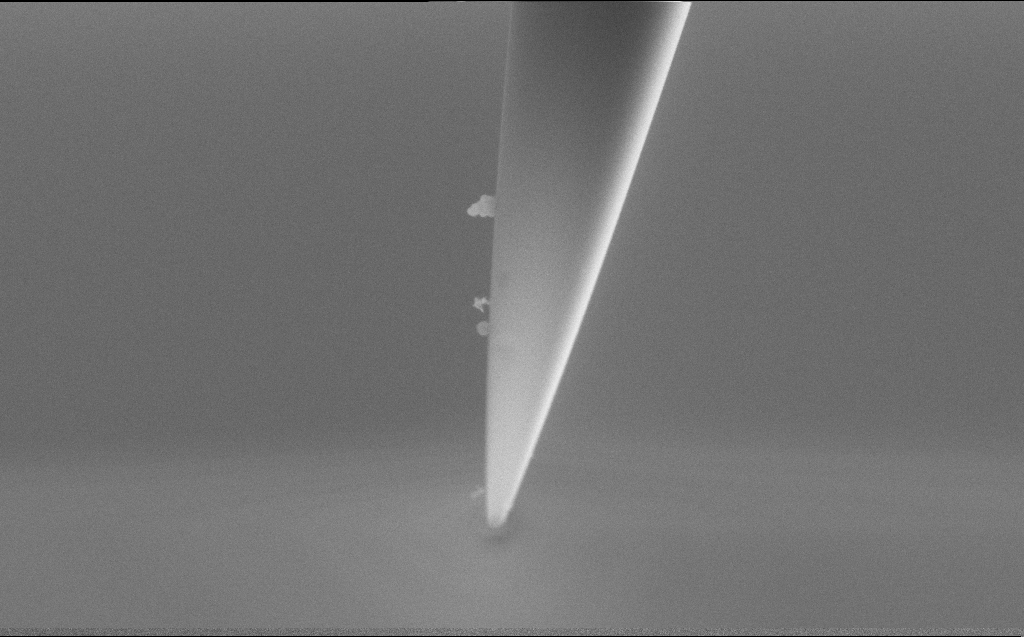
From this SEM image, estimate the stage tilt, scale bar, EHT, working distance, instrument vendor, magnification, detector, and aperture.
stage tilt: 45.1°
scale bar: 1000 nm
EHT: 5 kV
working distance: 5 mm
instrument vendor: Zeiss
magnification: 46.53 K X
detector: SE2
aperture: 30 µm